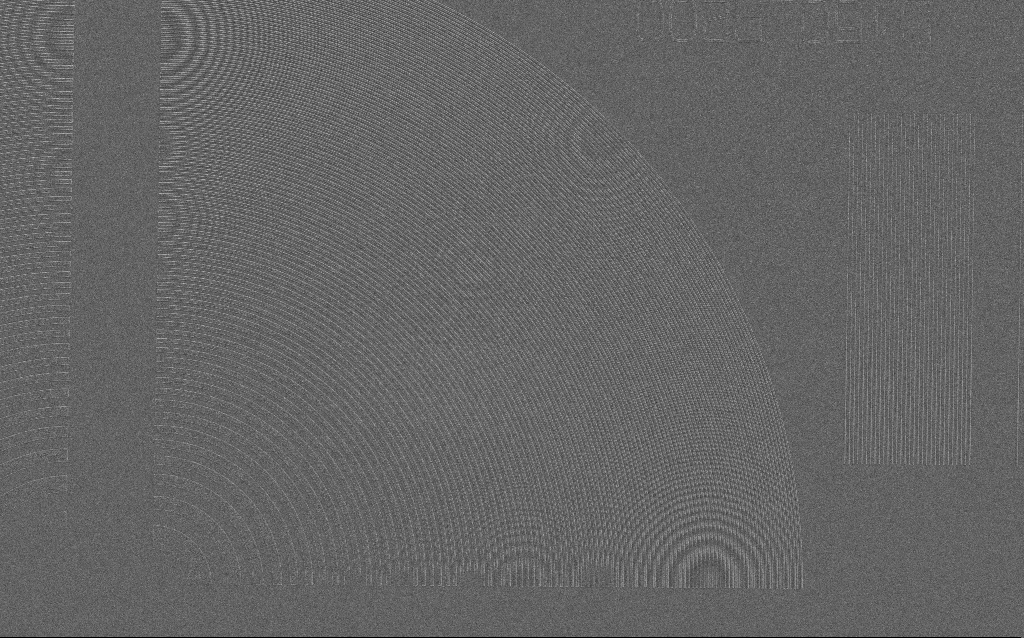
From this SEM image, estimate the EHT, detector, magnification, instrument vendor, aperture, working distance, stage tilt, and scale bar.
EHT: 1.5 kV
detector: SE2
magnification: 3.16 K X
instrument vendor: Zeiss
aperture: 30 µm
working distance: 6 mm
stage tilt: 0°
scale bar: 10000 nm